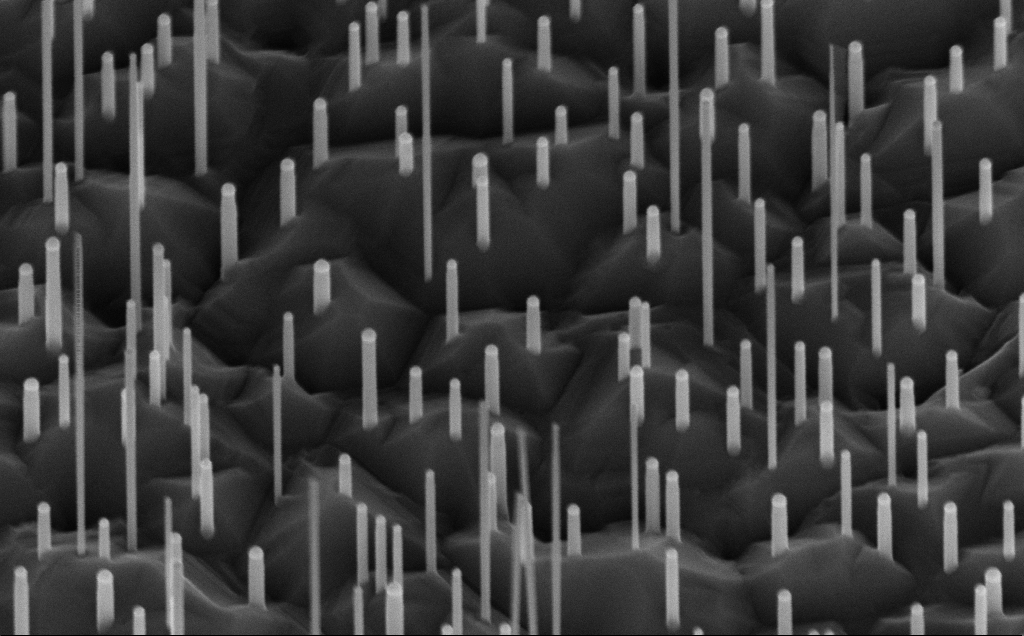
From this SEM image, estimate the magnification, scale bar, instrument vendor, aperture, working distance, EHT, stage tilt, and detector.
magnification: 80 K X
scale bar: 200 nm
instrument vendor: Zeiss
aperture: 30 µm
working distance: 7 mm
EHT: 10 kV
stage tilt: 45°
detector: InLens